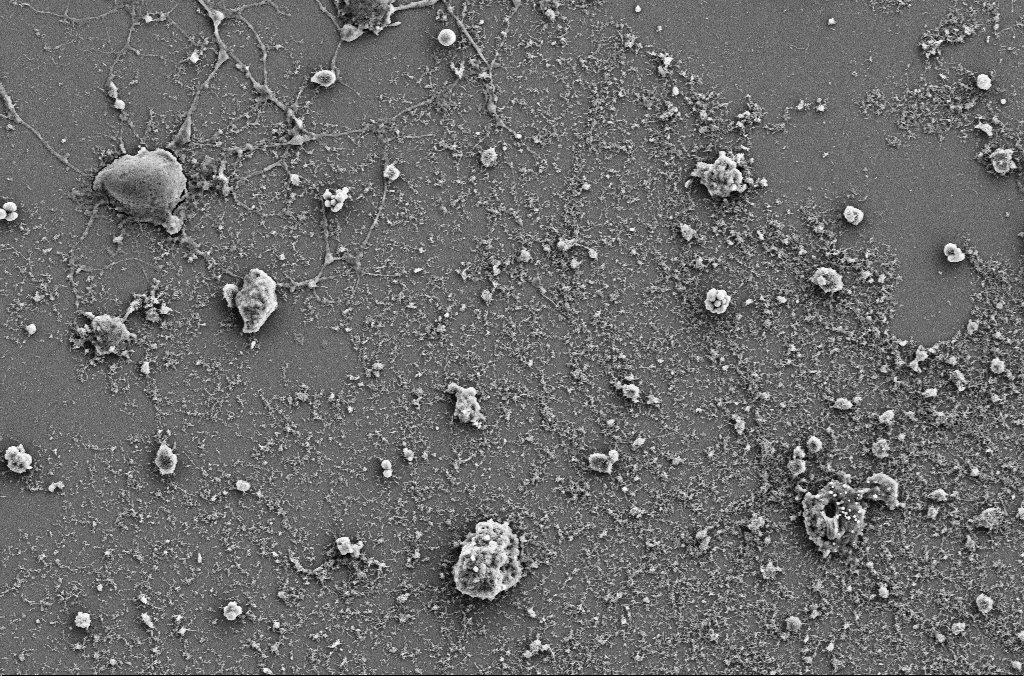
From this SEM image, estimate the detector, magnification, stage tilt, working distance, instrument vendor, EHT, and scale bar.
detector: SE2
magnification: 4 K X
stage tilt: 0°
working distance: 4 mm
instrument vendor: Zeiss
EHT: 5 kV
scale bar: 10000 nm